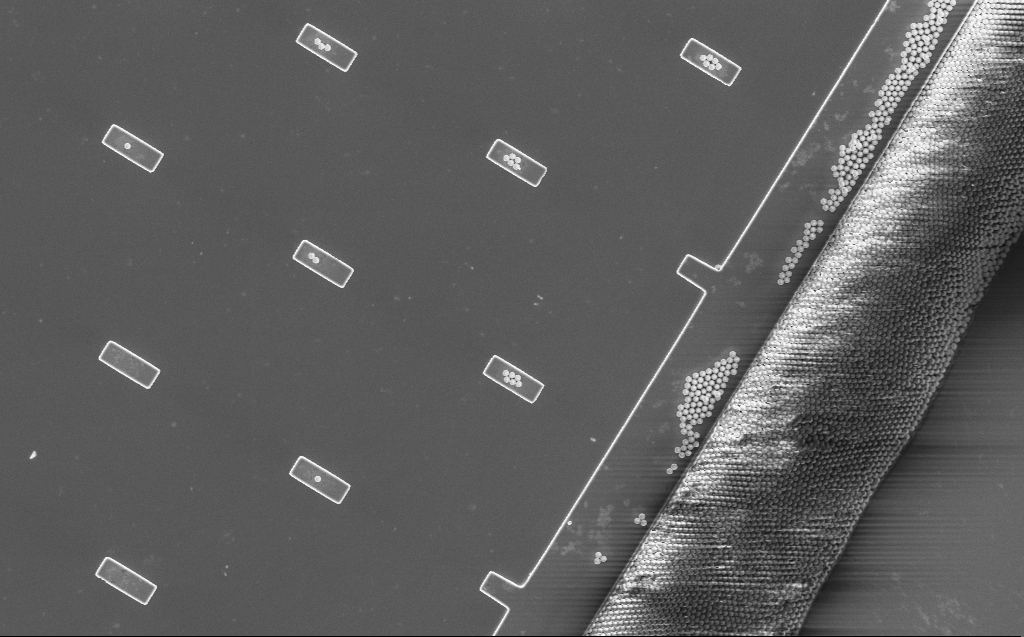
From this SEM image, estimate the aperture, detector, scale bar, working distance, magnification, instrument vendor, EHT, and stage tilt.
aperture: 30 µm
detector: InLens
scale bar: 10000 nm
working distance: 8 mm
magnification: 2.88 K X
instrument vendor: Zeiss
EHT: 5 kV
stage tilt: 0°